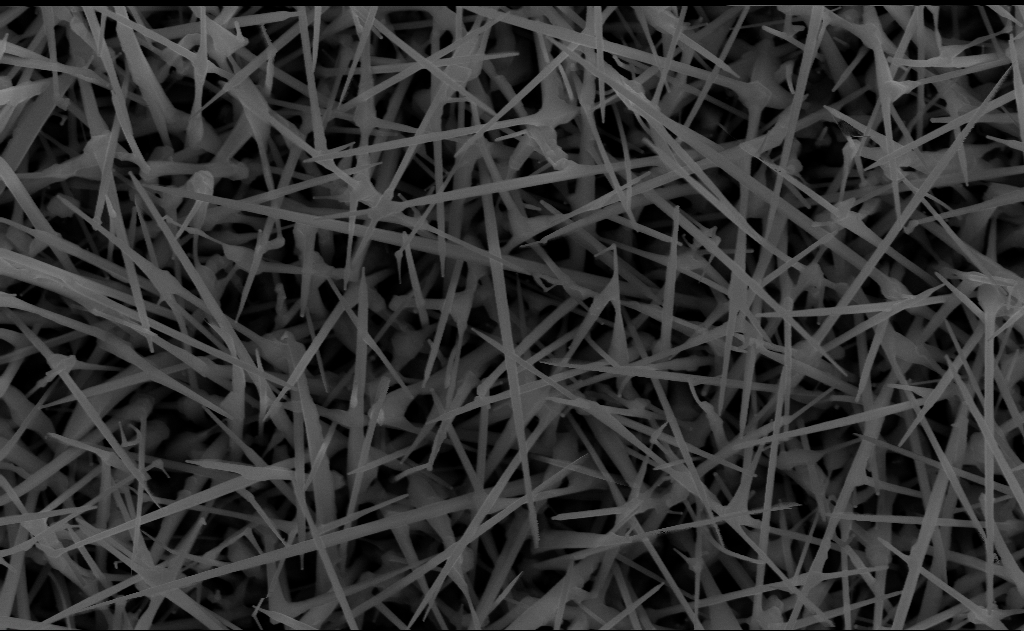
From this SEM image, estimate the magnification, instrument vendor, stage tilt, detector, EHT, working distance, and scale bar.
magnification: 40 K X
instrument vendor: Zeiss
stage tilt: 0°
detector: InLens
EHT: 10 kV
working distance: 10 mm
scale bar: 1000 nm